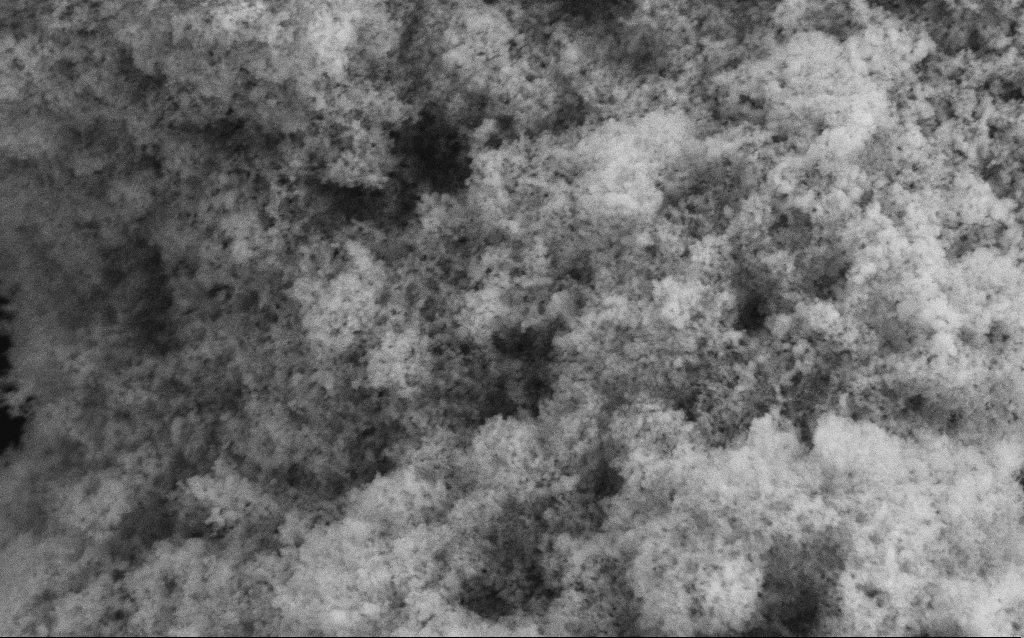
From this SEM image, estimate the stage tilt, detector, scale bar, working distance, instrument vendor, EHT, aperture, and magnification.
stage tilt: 0°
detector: SE2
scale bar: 1000 nm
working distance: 4.1 mm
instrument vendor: Zeiss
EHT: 5 kV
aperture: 30 µm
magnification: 68.64 K X